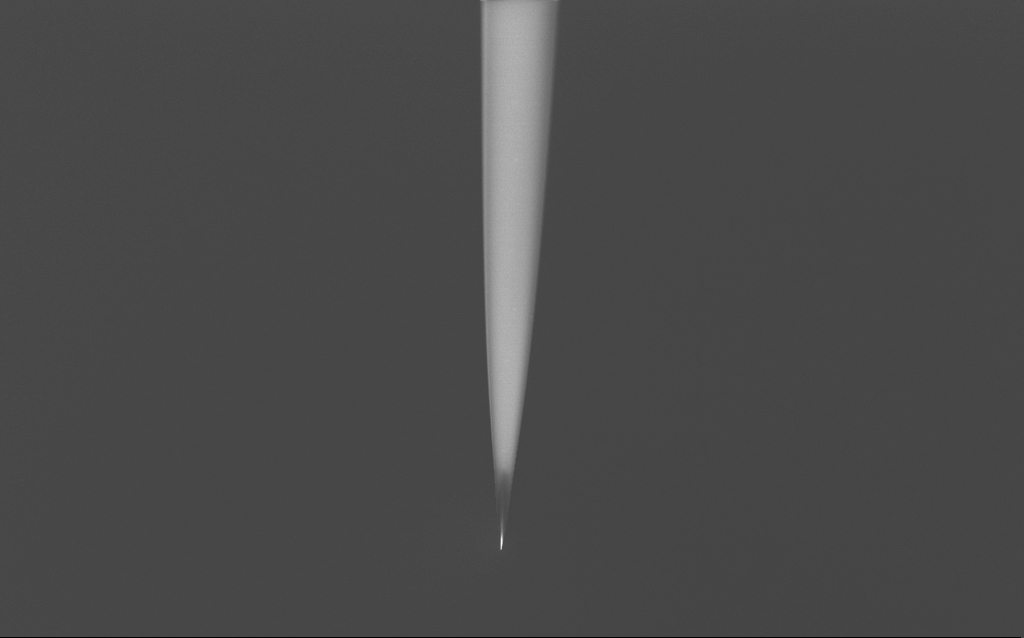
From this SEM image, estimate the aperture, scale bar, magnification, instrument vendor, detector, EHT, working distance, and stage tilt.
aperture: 30 µm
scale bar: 20000 nm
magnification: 1 K X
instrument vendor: Zeiss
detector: InLens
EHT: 2 kV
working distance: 6 mm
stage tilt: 45°